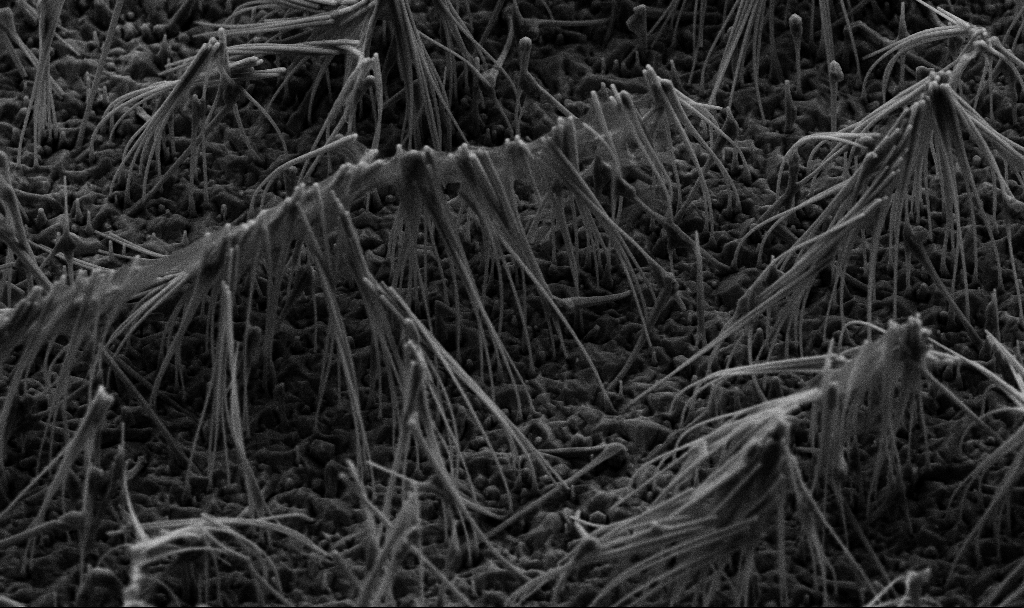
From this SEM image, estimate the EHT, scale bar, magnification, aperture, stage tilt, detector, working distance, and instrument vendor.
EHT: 5 kV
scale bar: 2000 nm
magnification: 13.8 K X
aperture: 30 µm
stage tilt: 45°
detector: SE2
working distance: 7.2 mm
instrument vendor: Zeiss